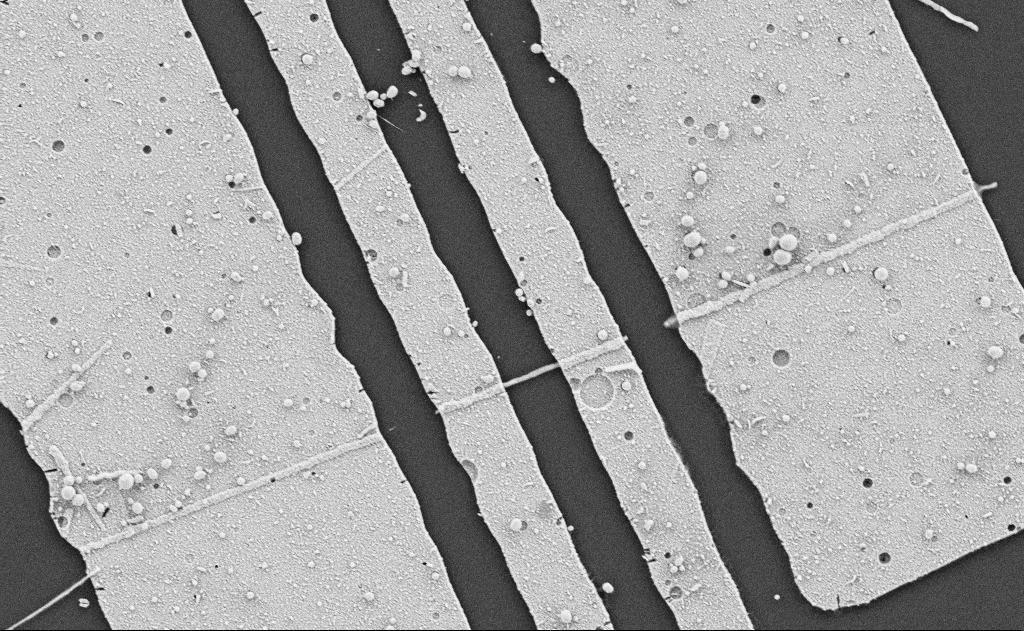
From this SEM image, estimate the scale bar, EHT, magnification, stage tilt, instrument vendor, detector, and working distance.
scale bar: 2000 nm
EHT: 5 kV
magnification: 11.84 K X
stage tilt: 0°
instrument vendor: Zeiss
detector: SE2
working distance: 7 mm